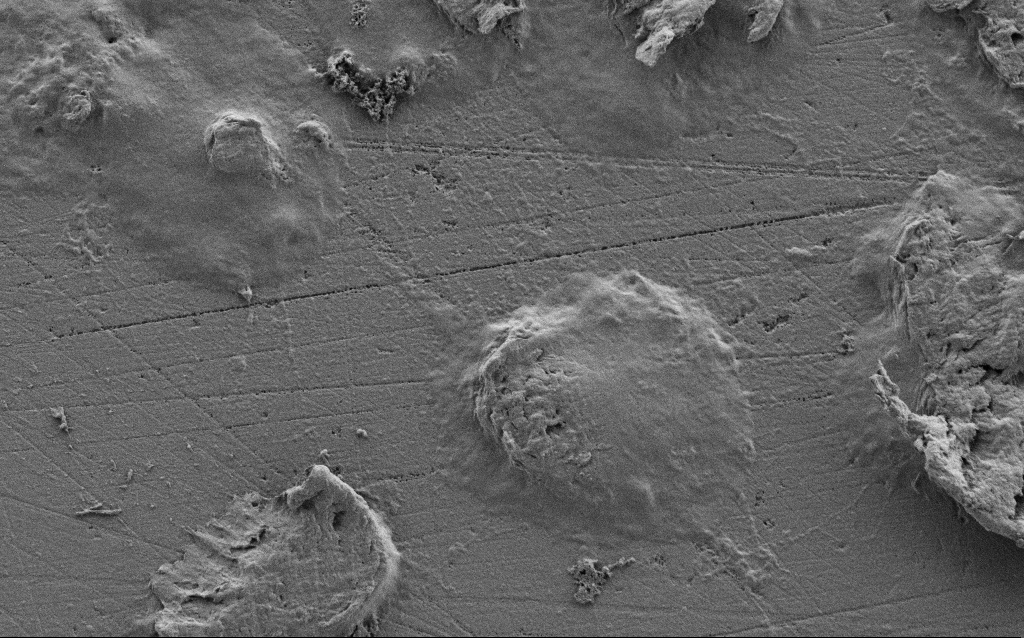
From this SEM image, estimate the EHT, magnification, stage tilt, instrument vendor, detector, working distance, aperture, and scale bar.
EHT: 1 kV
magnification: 8.64 K X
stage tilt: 0°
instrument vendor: Zeiss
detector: SE2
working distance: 5 mm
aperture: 30 µm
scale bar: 2000 nm